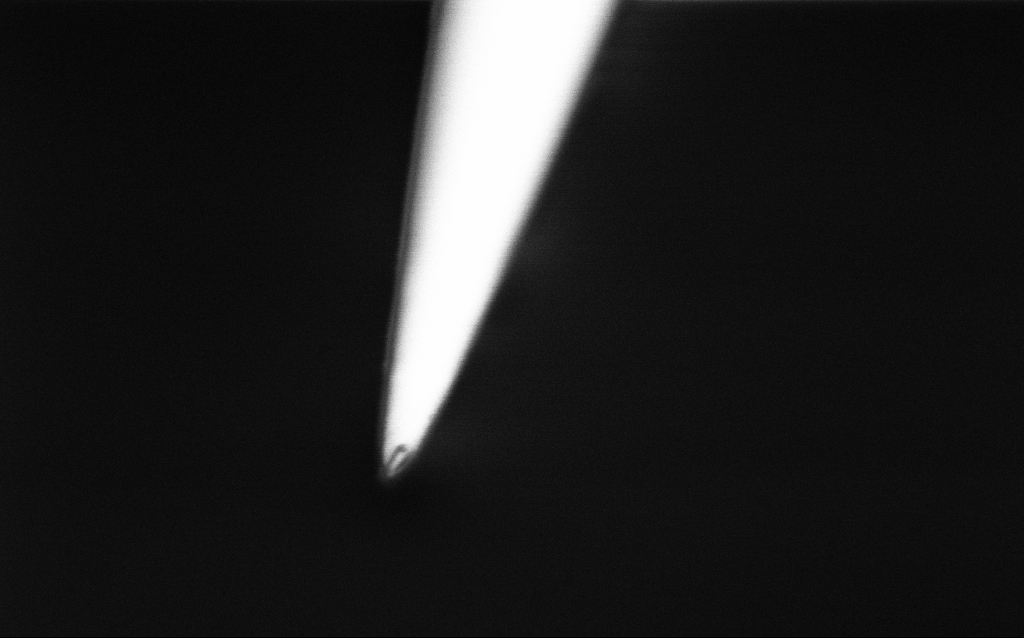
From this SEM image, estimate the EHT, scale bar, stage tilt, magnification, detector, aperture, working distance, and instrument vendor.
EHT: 1 kV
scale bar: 200 nm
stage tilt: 45°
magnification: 100 K X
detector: InLens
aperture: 30 µm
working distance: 6.9 mm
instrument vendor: Zeiss